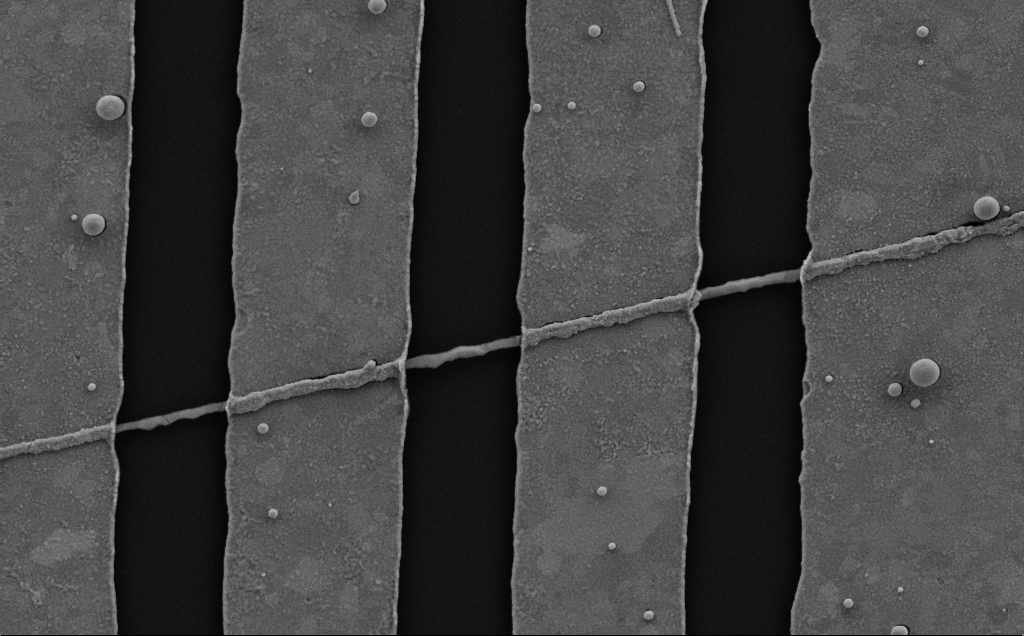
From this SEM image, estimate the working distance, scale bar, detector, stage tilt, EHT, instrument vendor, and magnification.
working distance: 6 mm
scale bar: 2000 nm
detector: SE2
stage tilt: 0°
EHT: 5 kV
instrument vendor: Zeiss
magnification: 26.67 K X